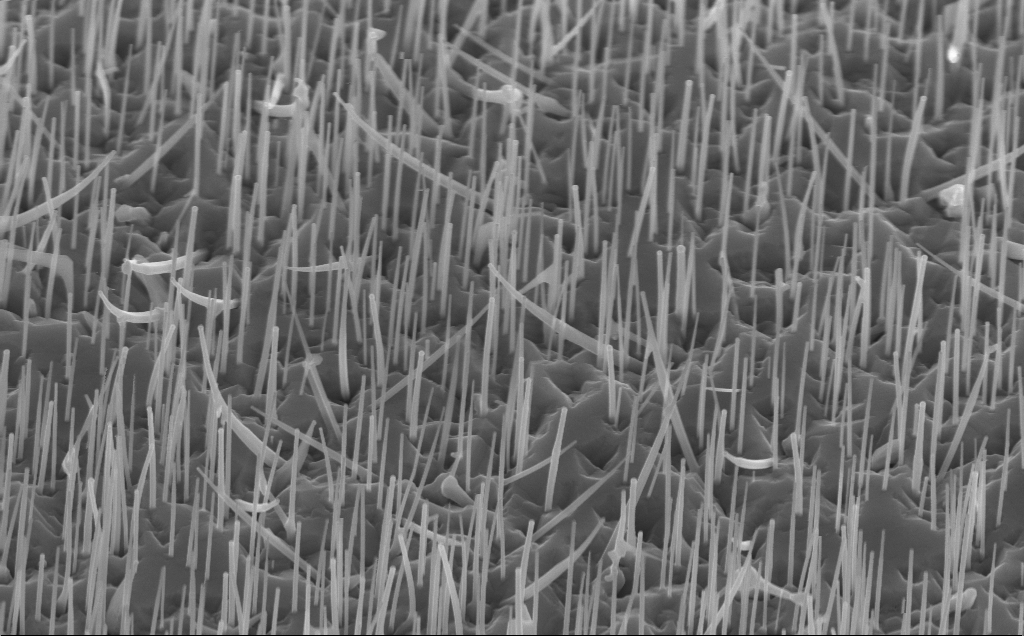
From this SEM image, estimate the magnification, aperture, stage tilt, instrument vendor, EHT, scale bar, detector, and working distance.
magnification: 40 K X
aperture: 30 µm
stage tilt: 45°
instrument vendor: Zeiss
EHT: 10 kV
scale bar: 1000 nm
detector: InLens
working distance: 5 mm